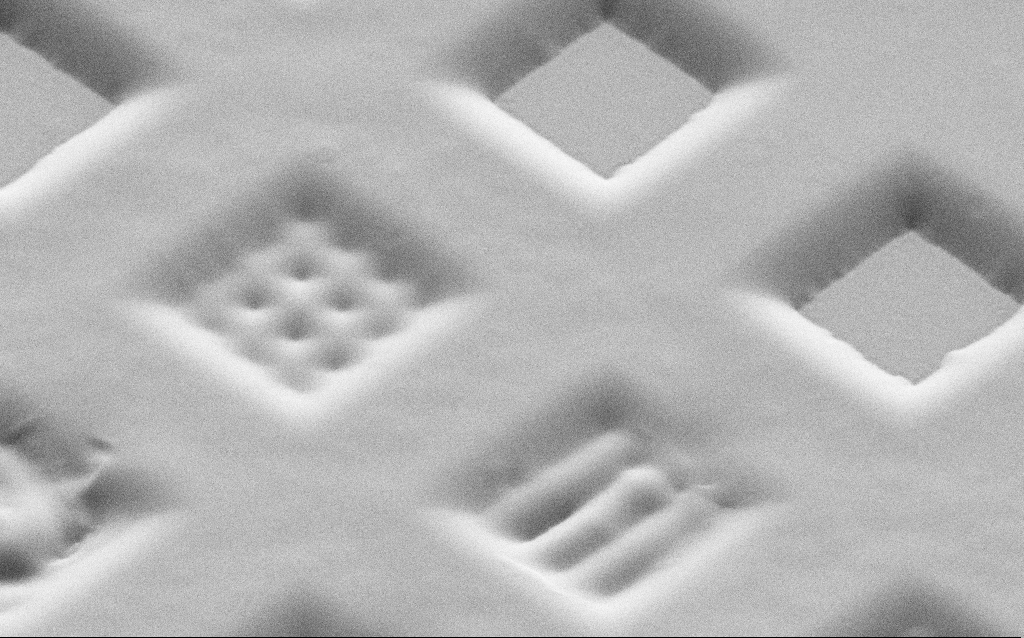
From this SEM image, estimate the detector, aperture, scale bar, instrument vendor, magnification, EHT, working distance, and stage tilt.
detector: SE2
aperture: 30 µm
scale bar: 20000 nm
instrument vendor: Zeiss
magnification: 1.11 K X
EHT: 1.5 kV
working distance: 6 mm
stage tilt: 45°